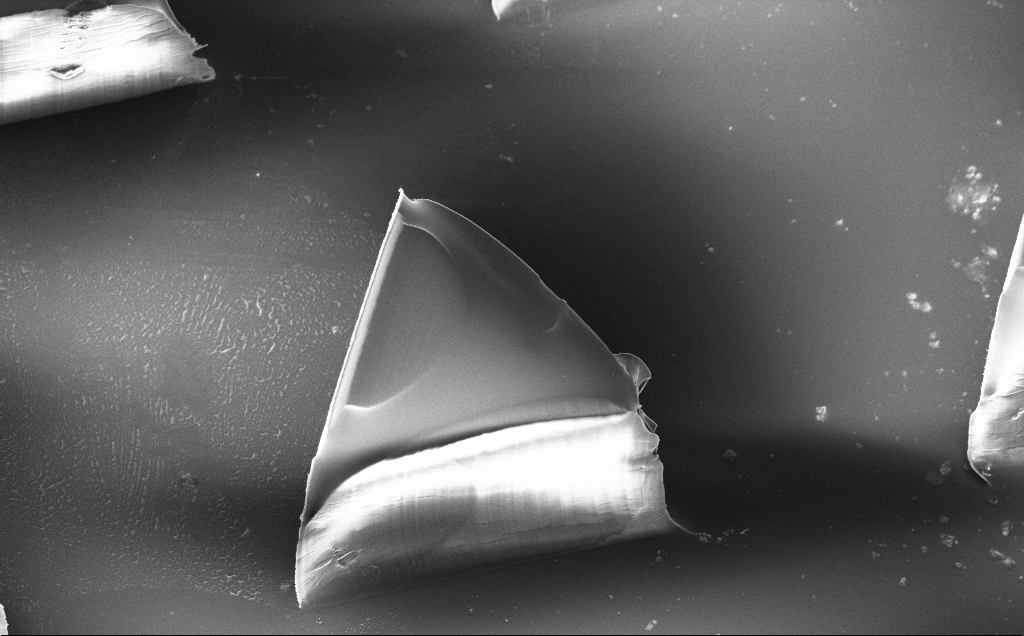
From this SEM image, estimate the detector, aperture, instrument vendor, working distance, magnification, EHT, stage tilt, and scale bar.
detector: InLens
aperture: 30 µm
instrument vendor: Zeiss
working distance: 9 mm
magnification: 0.548 K X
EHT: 10 kV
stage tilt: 20°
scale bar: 100000 nm